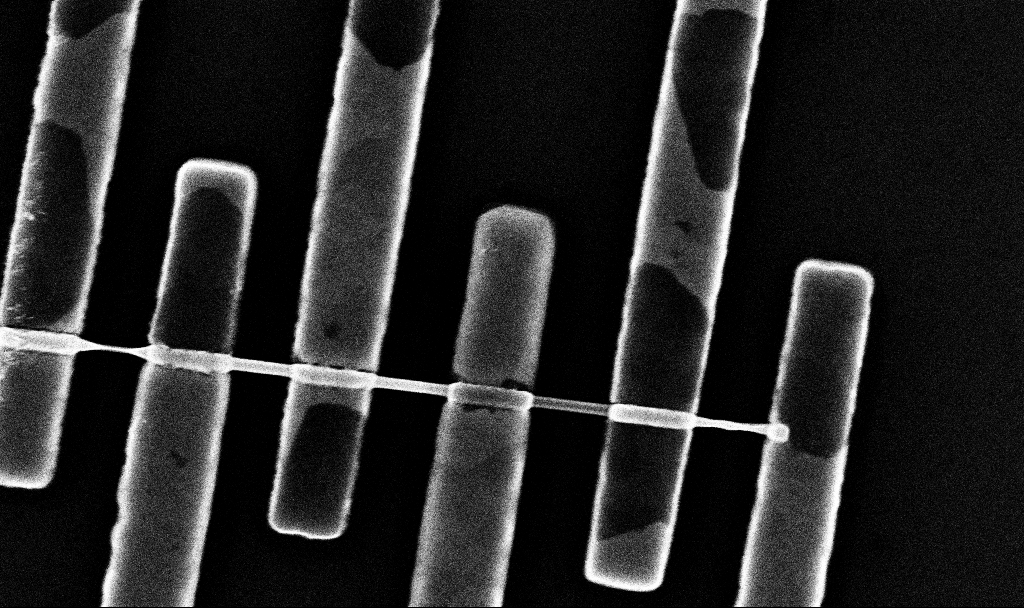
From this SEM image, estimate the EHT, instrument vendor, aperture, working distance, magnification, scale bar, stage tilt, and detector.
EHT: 10 kV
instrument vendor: Zeiss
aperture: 30 µm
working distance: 6.8 mm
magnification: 50.53 K X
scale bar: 1000 nm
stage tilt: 0°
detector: InLens